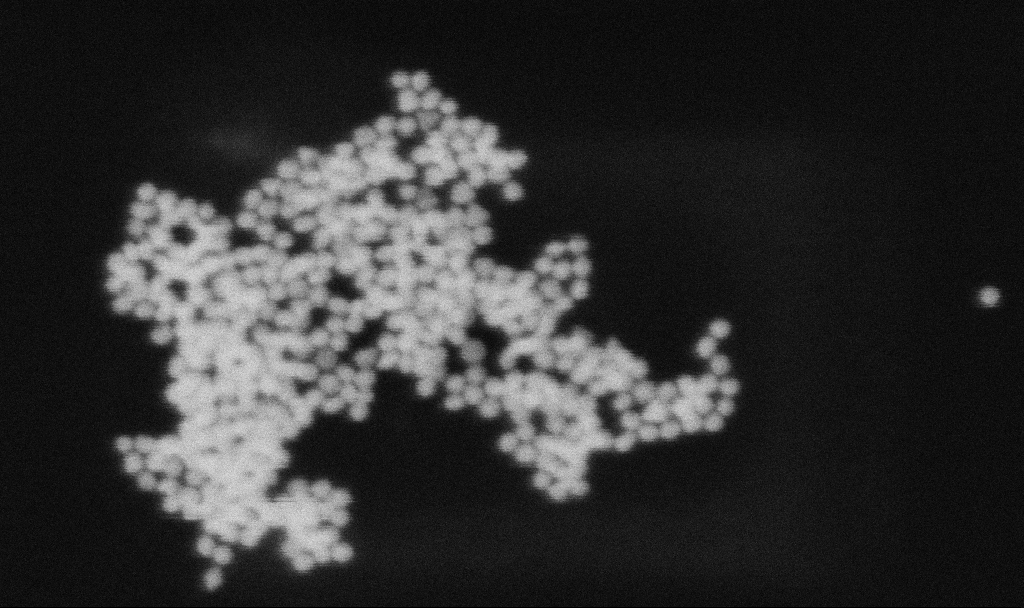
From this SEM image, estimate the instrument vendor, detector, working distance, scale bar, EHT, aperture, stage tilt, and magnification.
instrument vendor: Zeiss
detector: SE2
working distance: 11.3 mm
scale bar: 100 nm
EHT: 20 kV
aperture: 30 µm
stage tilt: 0°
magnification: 400 K X